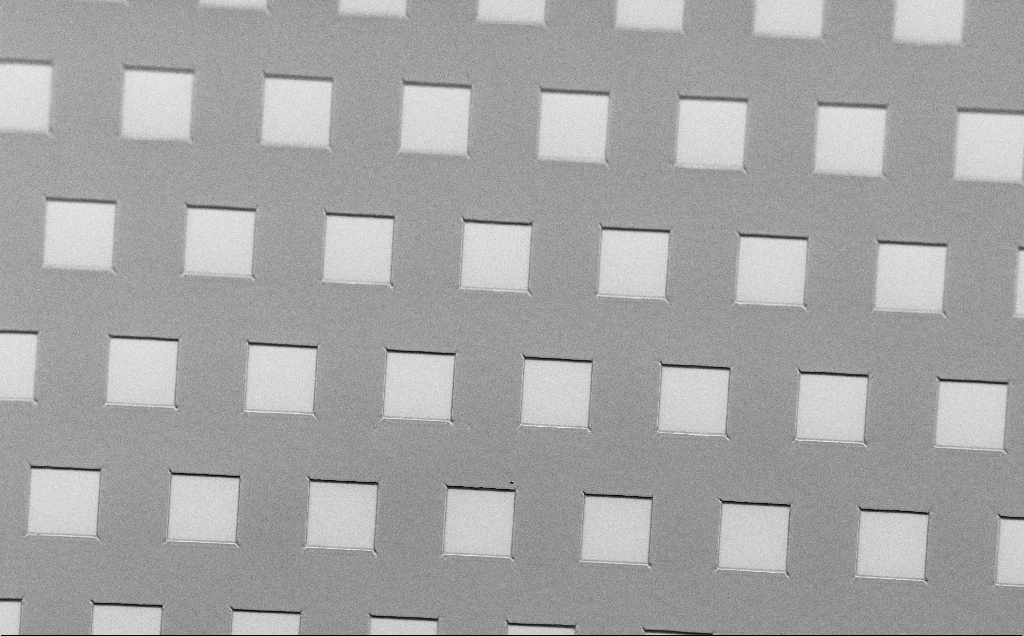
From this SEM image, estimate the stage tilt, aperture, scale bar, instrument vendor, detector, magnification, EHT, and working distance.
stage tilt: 45°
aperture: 30 µm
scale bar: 100000 nm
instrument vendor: Zeiss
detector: SE2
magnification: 0.505 K X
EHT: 1.5 kV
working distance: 7 mm